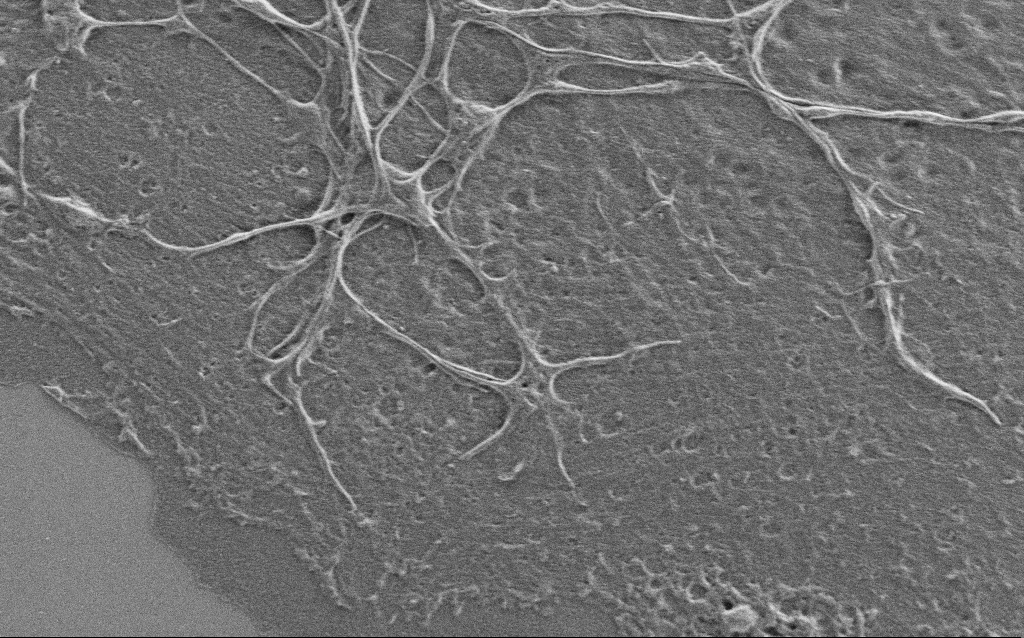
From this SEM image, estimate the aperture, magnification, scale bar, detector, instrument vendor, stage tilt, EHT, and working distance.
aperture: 30 µm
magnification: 10 K X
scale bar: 2000 nm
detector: SE2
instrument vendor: Zeiss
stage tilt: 0°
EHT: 0.9 kV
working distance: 4 mm